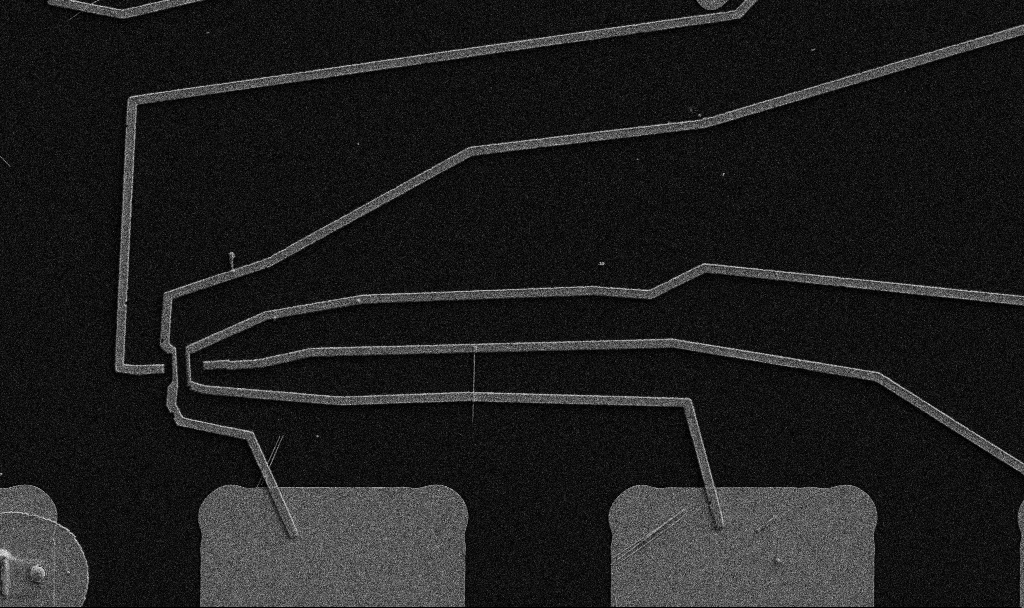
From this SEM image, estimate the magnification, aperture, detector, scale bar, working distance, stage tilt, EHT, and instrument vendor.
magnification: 5 K X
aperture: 30 µm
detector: SE2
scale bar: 10000 nm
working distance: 10.7 mm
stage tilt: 0°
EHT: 5 kV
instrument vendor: Zeiss